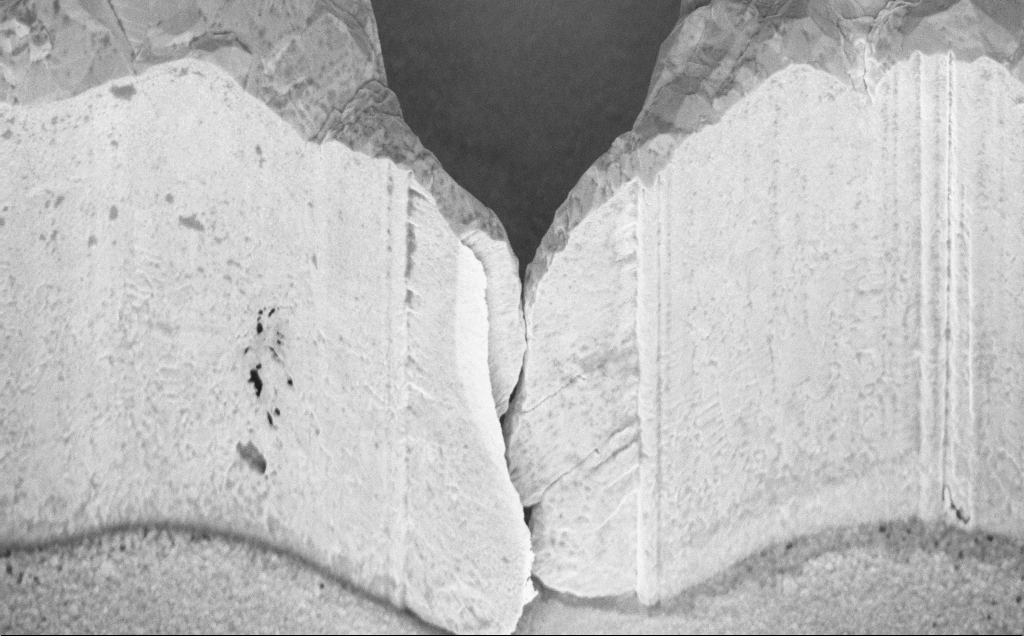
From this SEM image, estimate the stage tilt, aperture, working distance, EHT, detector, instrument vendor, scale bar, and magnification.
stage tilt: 45°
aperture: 30 µm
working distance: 9 mm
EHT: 5 kV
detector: InLens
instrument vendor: Zeiss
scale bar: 1000 nm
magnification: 24.54 K X